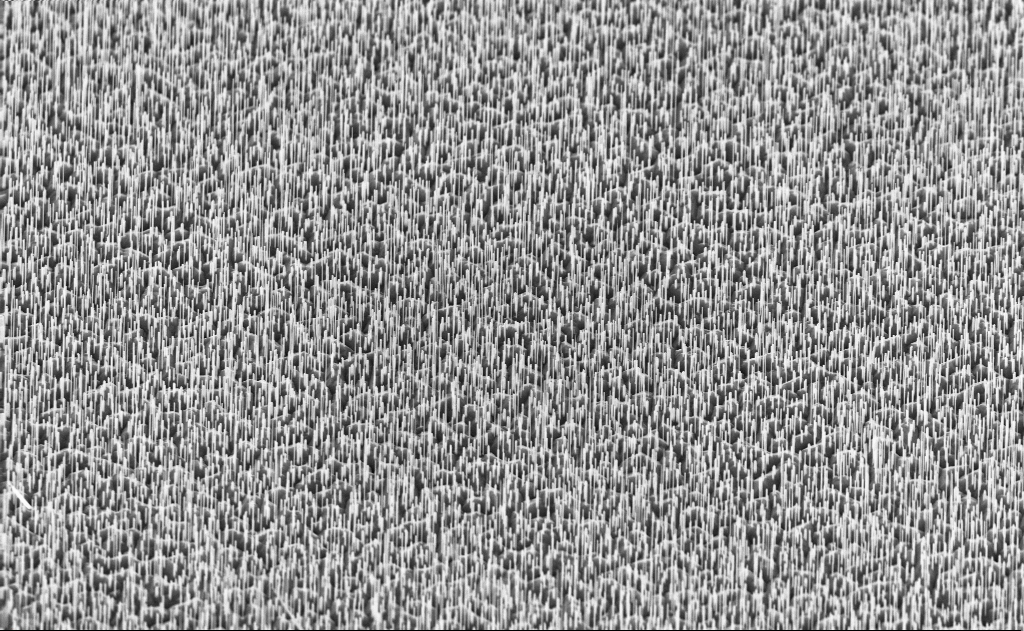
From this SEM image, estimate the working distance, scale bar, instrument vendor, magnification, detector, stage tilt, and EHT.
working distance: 6 mm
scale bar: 2000 nm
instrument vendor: Zeiss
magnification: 10 K X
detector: InLens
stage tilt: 45°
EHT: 10 kV